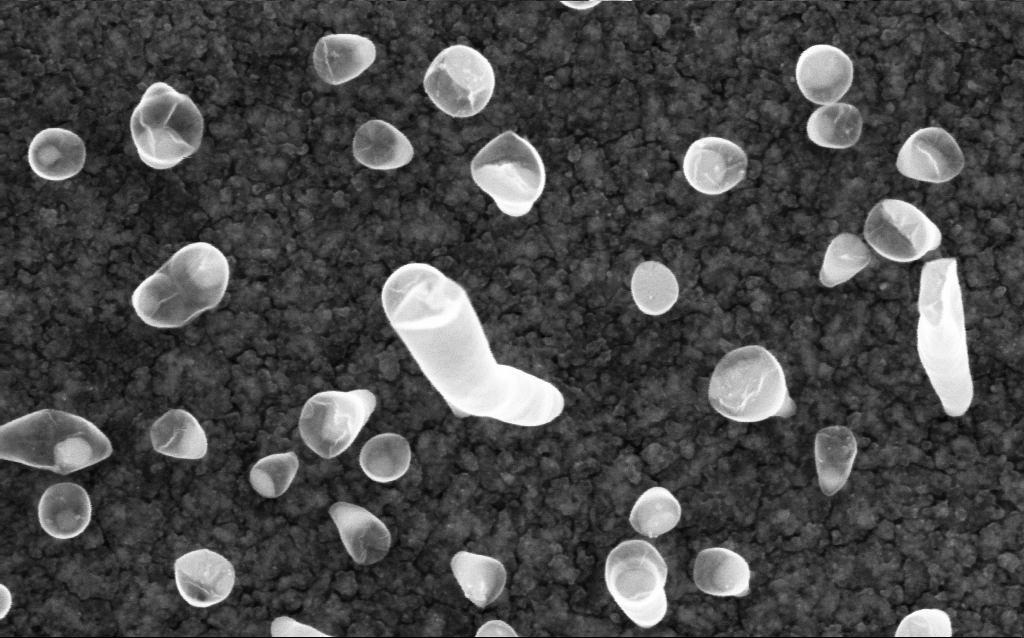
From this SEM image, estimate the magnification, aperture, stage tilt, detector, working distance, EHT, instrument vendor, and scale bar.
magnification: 200 K X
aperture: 30 µm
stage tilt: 0°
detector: InLens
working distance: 2 mm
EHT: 5 kV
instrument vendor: Zeiss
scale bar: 100 nm